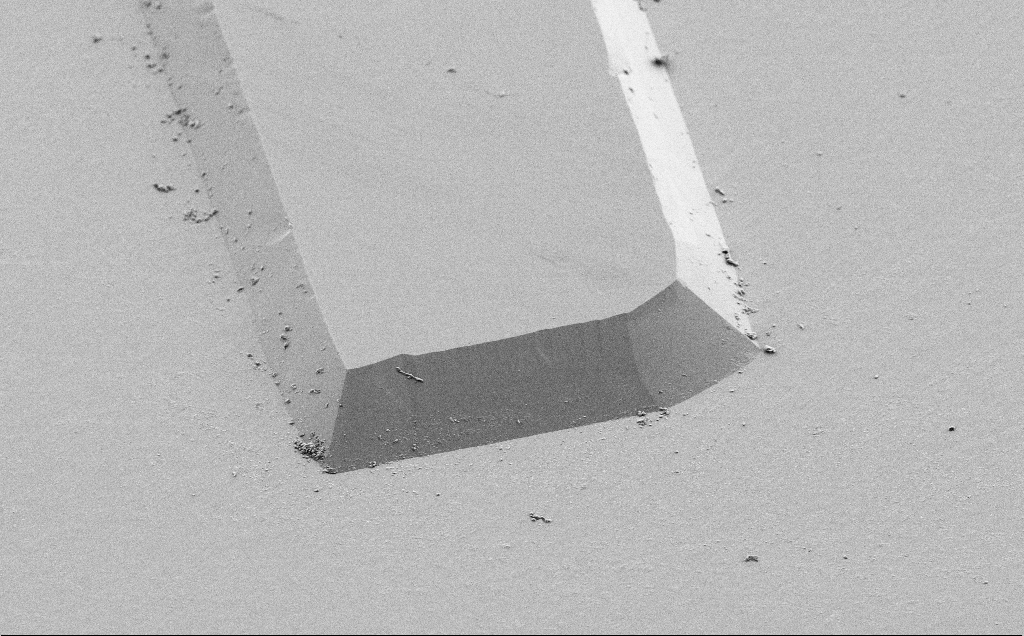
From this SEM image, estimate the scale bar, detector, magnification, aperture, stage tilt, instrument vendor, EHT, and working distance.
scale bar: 20000 nm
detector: SE2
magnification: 1.87 K X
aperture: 30 µm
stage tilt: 45°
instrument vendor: Zeiss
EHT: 3 kV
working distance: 9 mm